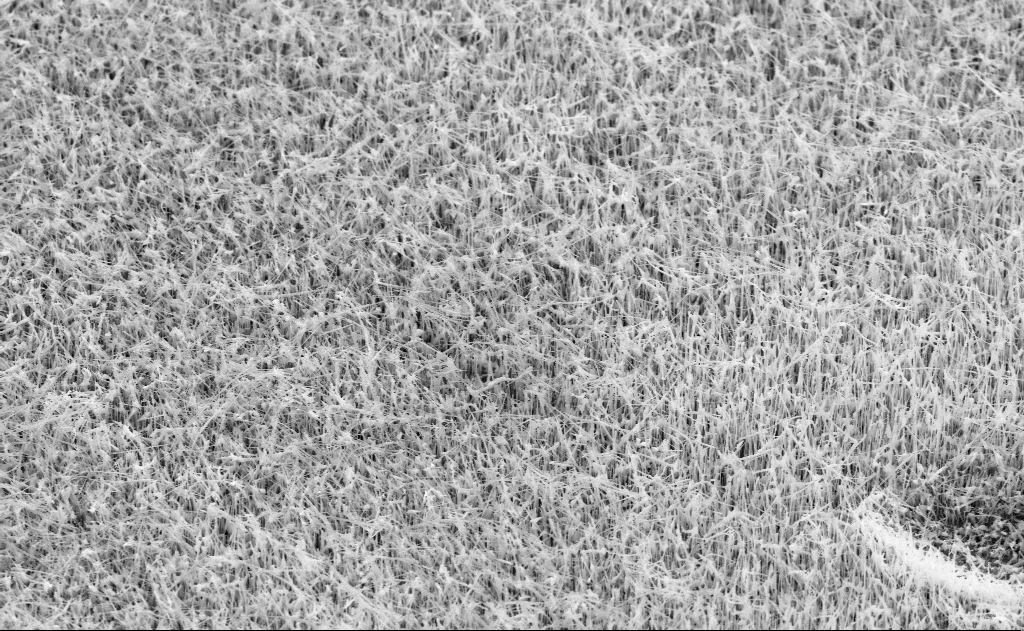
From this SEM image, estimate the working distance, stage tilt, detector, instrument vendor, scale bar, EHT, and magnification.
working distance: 14 mm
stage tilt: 45°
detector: SE2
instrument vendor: Zeiss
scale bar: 2000 nm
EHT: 10 kV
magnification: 10 K X